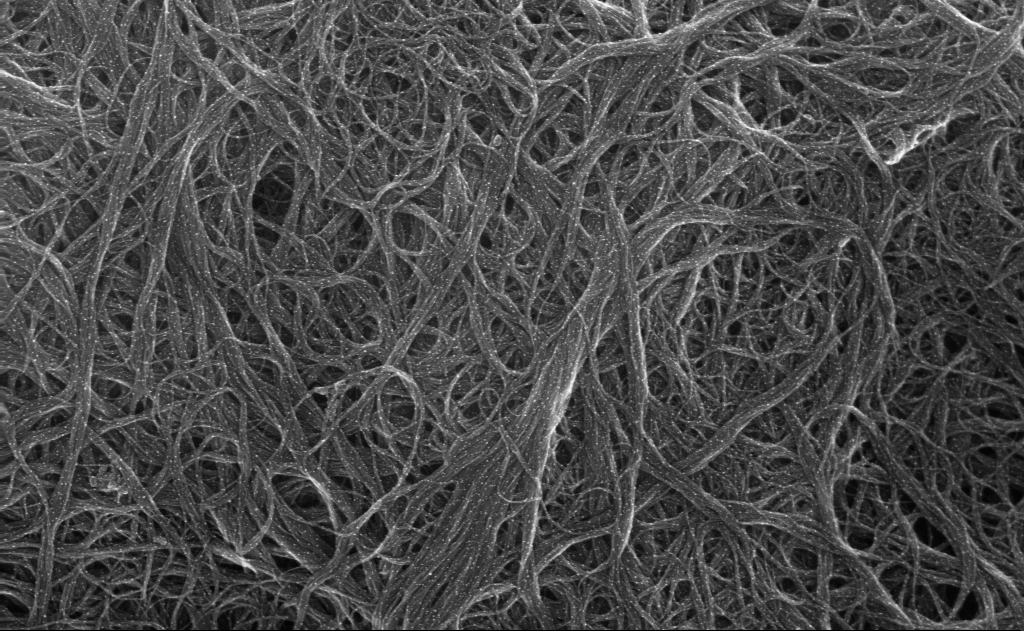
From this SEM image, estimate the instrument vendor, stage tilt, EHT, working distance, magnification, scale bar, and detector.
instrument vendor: Zeiss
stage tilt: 0°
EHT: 10 kV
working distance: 3 mm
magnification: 112.52 K X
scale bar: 200 nm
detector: InLens